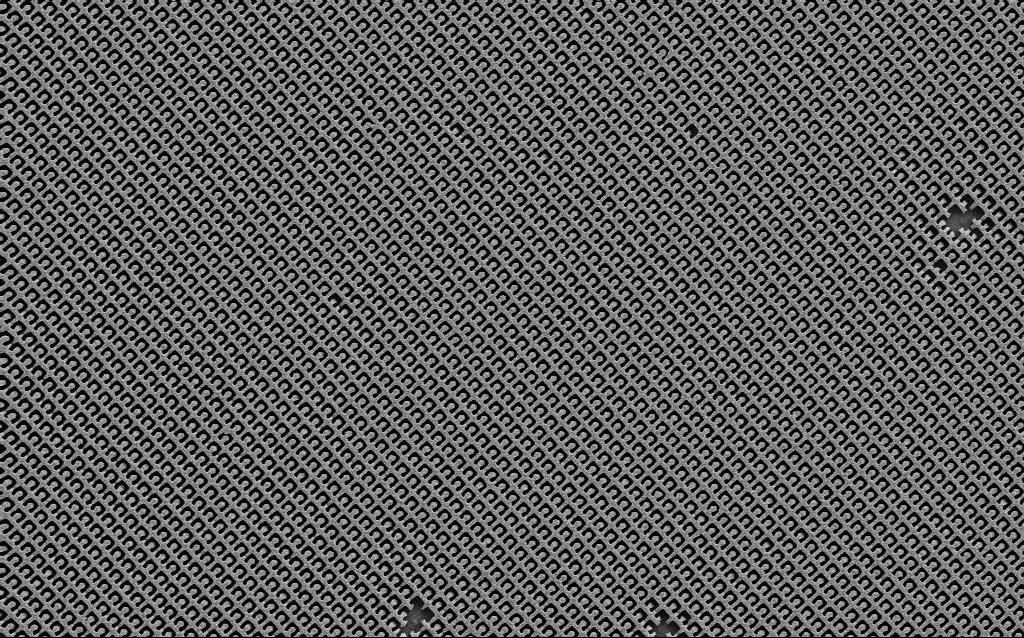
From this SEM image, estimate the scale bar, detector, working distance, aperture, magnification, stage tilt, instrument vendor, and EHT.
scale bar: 2000 nm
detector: SE2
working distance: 6.1 mm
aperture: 30 µm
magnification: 12.61 K X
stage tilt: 0°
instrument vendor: Zeiss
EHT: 5 kV